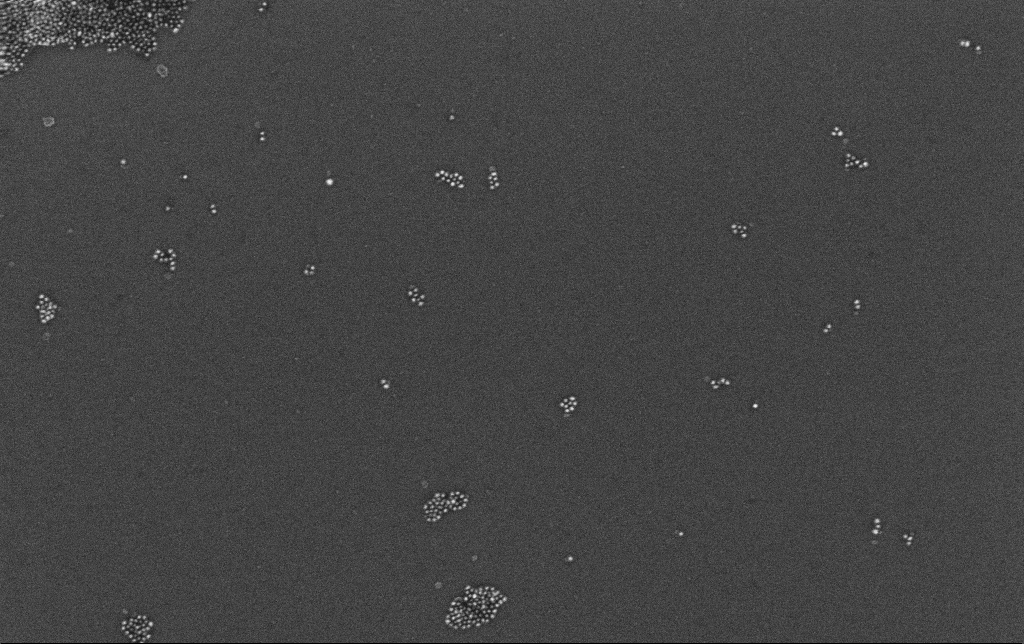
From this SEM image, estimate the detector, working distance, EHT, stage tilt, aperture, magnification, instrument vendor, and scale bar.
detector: InLens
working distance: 3.4 mm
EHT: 10 kV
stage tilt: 0°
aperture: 30 µm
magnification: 100 K X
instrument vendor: Zeiss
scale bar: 200 nm